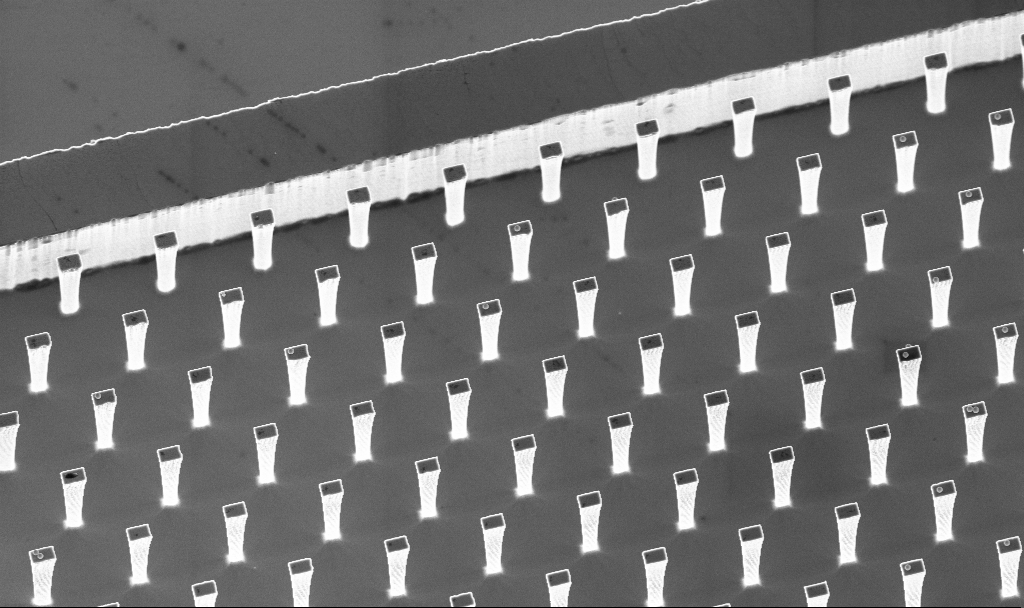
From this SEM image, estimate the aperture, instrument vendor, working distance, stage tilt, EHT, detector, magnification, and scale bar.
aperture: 30 µm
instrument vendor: Zeiss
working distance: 4.7 mm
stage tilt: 20°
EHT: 5 kV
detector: InLens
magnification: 3.04 K X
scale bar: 20000 nm